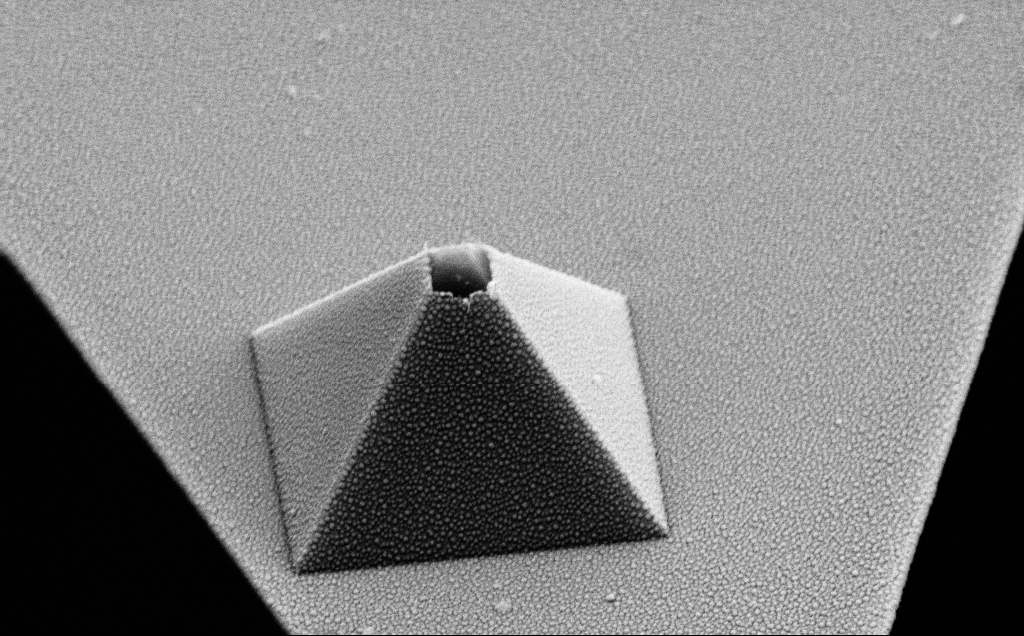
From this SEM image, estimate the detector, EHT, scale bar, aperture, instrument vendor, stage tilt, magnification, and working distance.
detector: SE2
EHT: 10 kV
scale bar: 2000 nm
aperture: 30 µm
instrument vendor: Zeiss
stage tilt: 45°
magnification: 26.79 K X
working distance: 8 mm